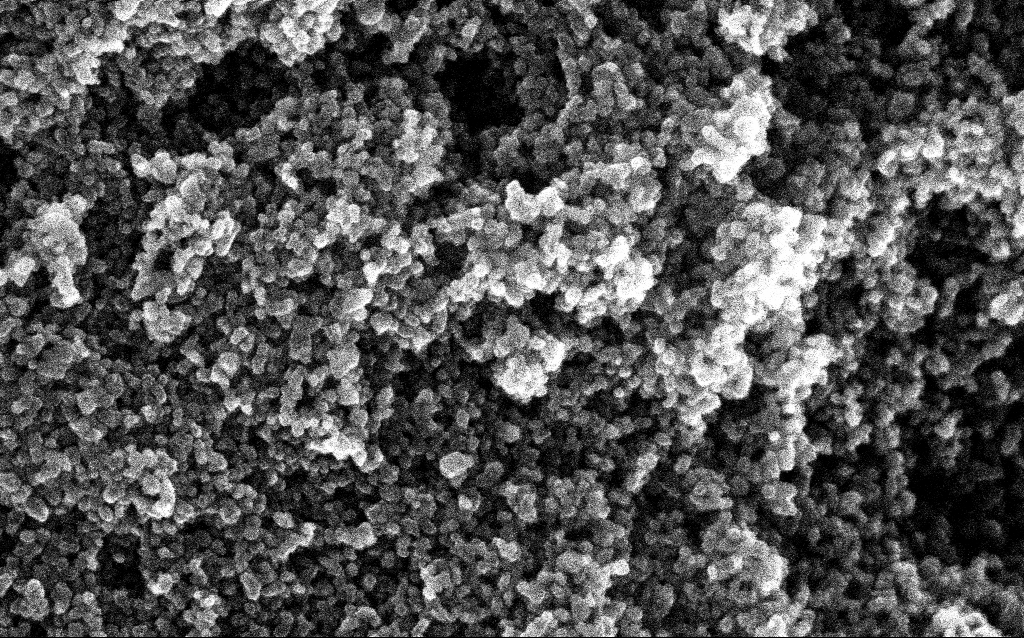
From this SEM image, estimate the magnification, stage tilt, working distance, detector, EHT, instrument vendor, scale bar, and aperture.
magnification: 204.13 K X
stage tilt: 0°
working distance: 2.8 mm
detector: InLens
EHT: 10 kV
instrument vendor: Zeiss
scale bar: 100 nm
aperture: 30 µm